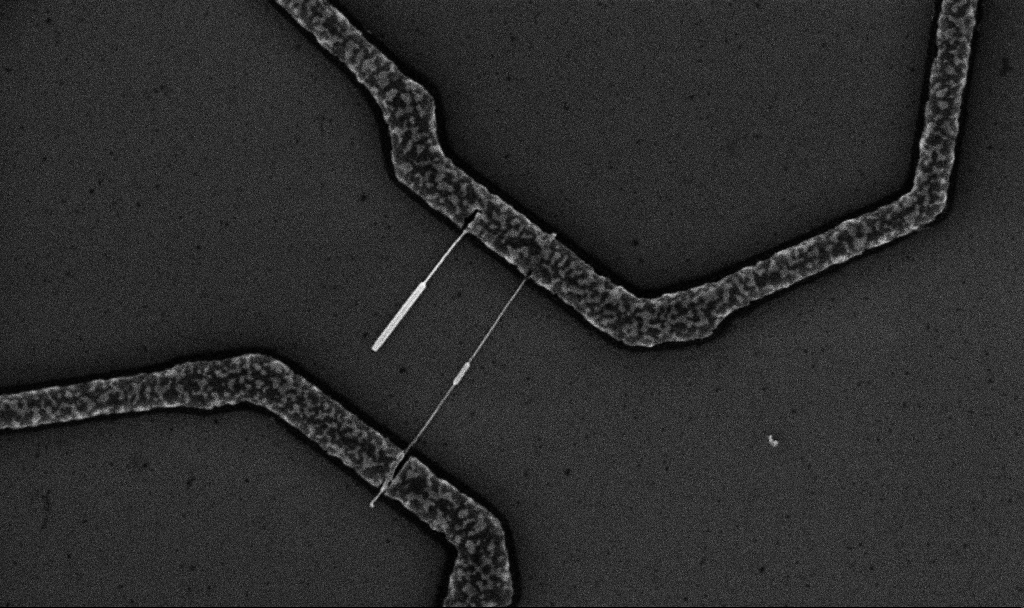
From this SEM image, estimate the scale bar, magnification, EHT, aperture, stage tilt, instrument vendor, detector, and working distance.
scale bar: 1000 nm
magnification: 20 K X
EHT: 10 kV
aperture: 30 µm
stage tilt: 0°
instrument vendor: Zeiss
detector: InLens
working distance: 6.7 mm